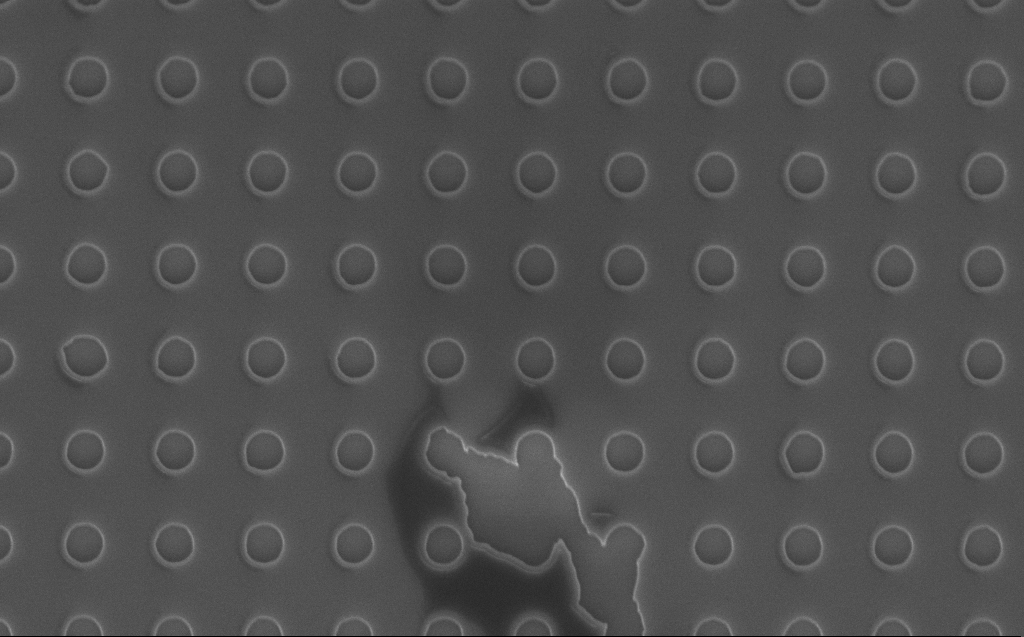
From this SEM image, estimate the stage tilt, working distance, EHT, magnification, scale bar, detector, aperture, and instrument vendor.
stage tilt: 45°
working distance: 7 mm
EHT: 5 kV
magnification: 31.49 K X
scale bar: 1000 nm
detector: InLens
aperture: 30 µm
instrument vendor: Zeiss